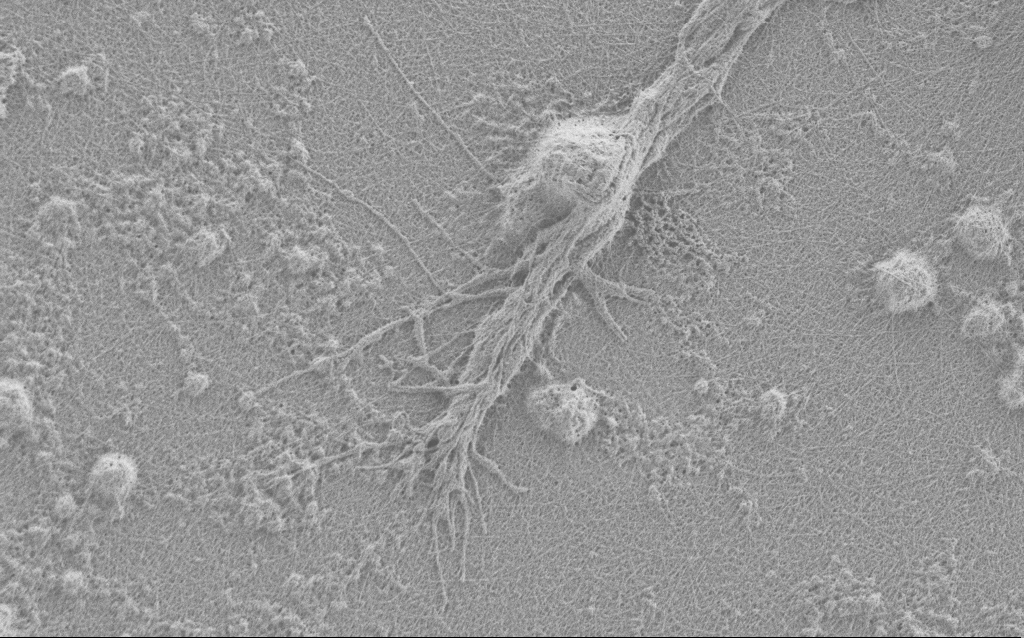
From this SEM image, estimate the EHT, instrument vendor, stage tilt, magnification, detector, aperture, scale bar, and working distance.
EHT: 0.9 kV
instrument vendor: Zeiss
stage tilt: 0°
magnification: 10 K X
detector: SE2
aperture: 30 µm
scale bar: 2000 nm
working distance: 4 mm